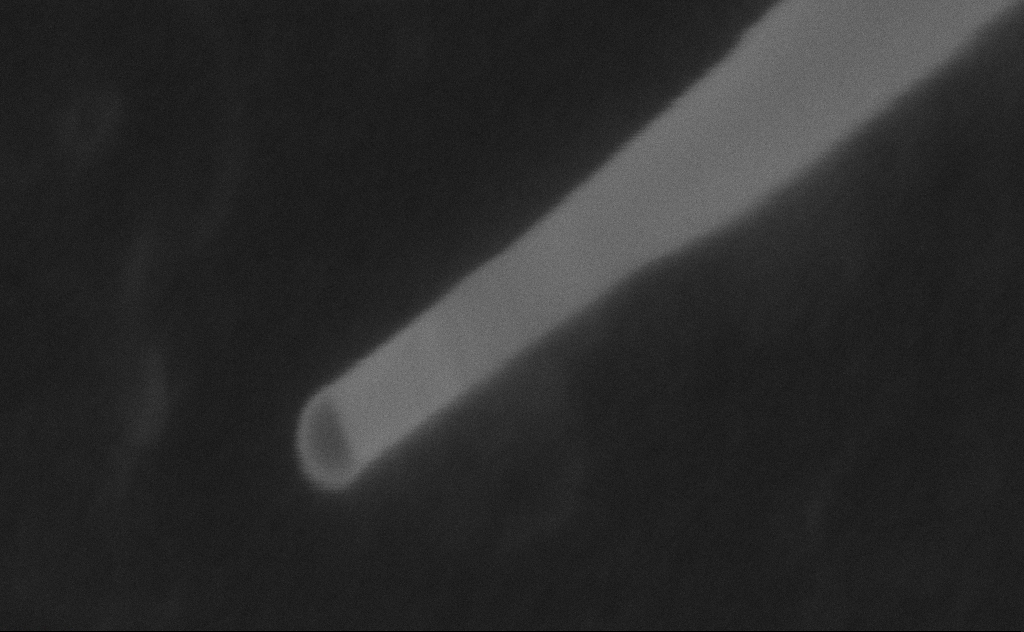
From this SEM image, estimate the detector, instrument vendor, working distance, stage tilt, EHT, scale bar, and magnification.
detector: SE2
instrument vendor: Zeiss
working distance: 9 mm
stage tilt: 0°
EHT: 20 kV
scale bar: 100 nm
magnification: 661.99 K X